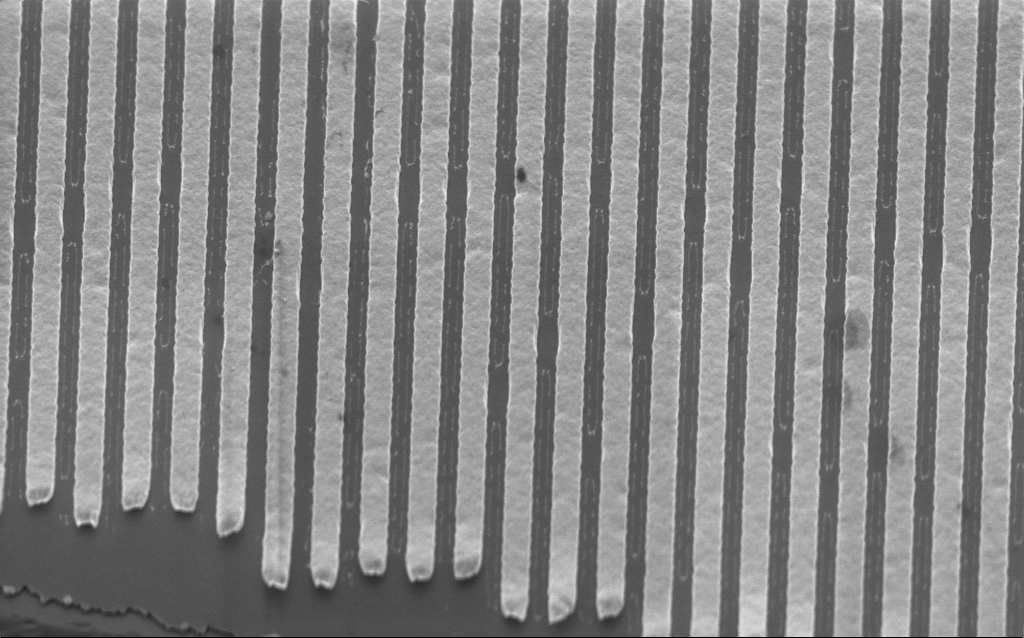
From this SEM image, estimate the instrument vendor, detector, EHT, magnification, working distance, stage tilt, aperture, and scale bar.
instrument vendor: Zeiss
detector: InLens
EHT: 2 kV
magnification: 35.29 K X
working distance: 3.4 mm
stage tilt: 45°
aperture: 30 µm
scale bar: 1000 nm